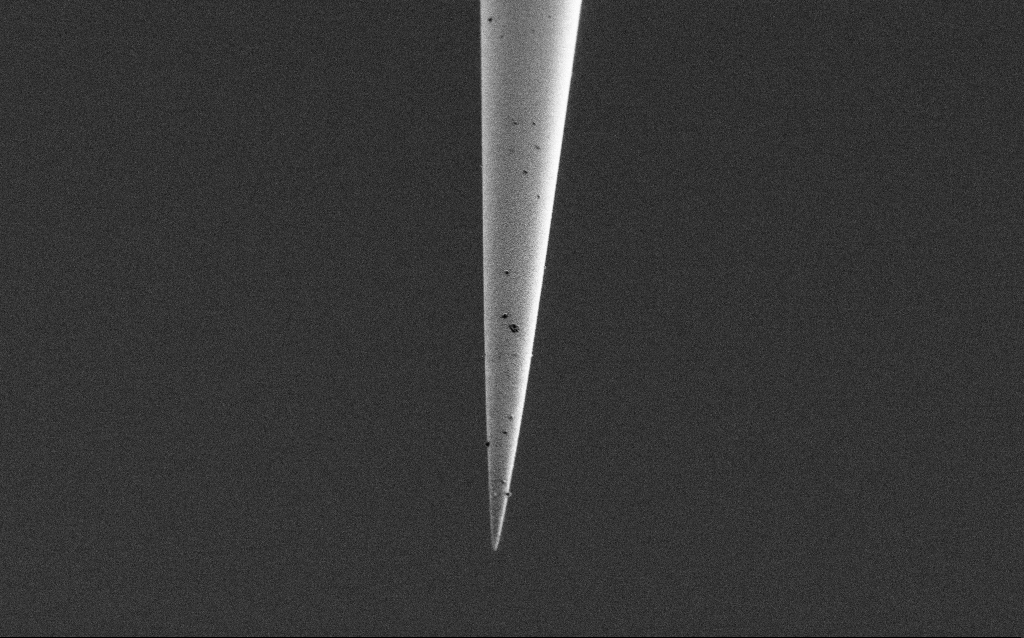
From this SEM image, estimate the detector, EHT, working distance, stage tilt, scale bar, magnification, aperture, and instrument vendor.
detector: SE2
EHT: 1 kV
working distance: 6.7 mm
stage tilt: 45°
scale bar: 2000 nm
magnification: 10 K X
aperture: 30 µm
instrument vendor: Zeiss